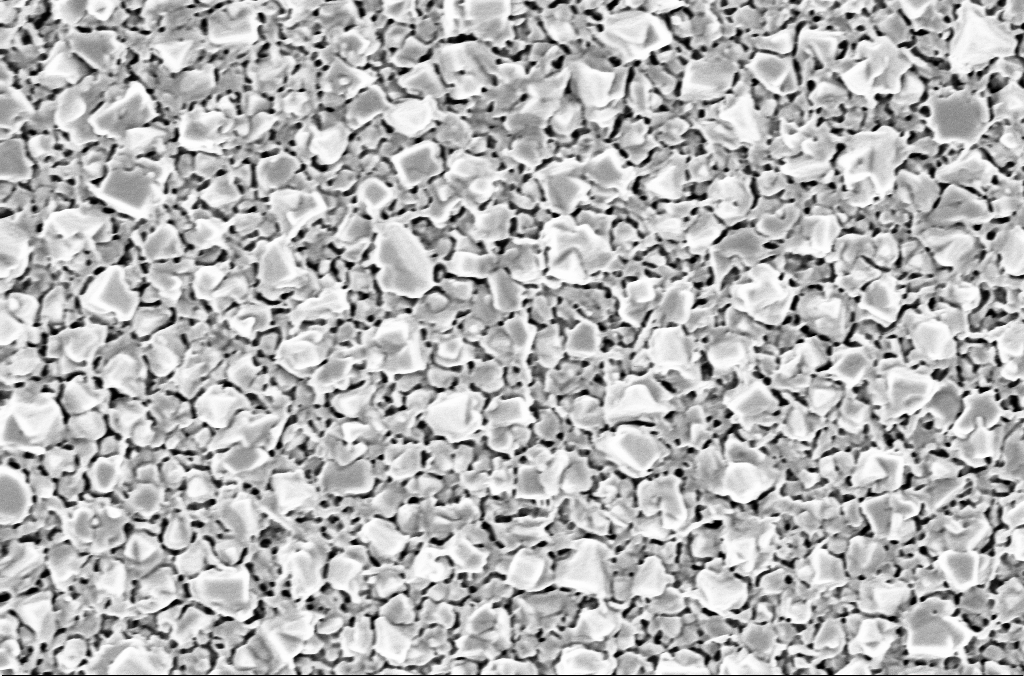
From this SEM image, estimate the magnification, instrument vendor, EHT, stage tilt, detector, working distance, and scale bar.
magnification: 50 K X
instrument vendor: Zeiss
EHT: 2 kV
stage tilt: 0°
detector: InLens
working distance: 1.9 mm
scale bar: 1000 nm